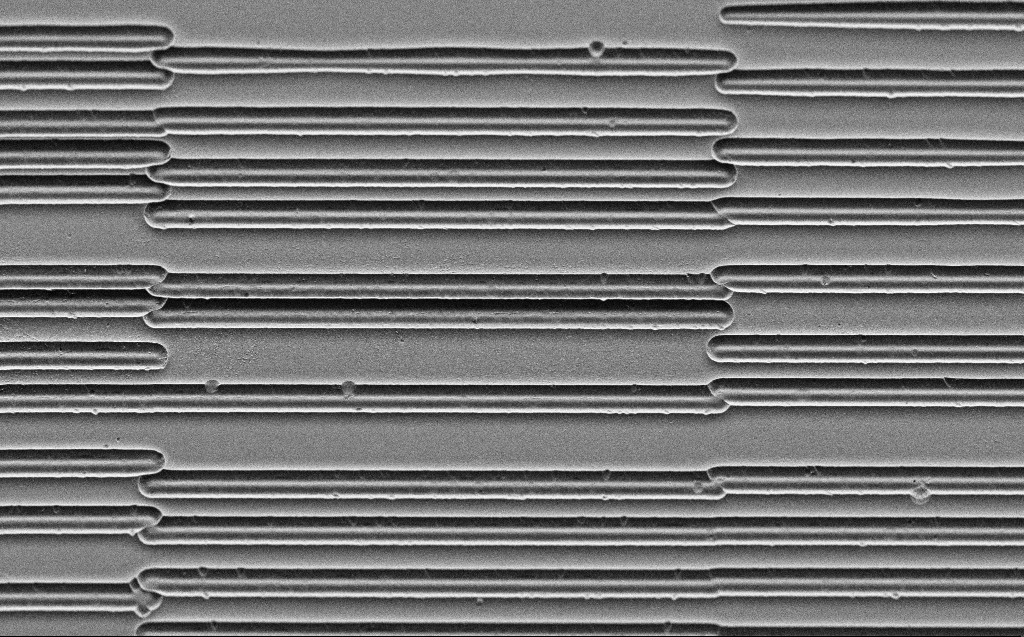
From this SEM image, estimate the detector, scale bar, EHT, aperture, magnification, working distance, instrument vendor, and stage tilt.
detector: SE2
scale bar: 20000 nm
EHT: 3 kV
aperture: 30 µm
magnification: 2.13 K X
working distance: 6 mm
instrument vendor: Zeiss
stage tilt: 45°